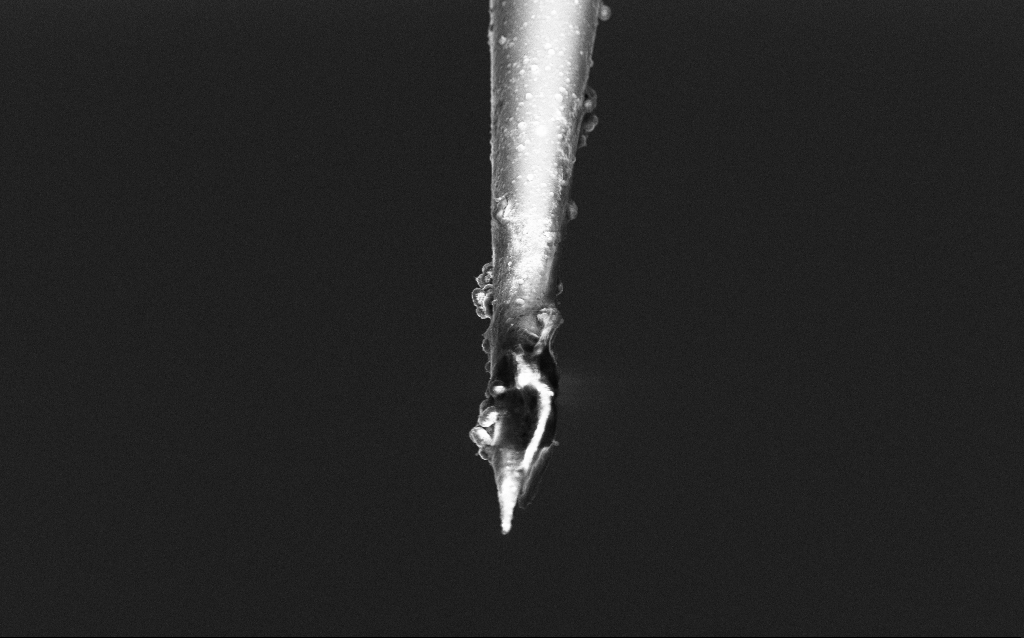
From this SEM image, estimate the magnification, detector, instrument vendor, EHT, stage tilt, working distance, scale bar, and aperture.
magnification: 5 K X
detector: InLens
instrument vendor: Zeiss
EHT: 5 kV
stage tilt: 43.9°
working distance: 6 mm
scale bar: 10000 nm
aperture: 30 µm